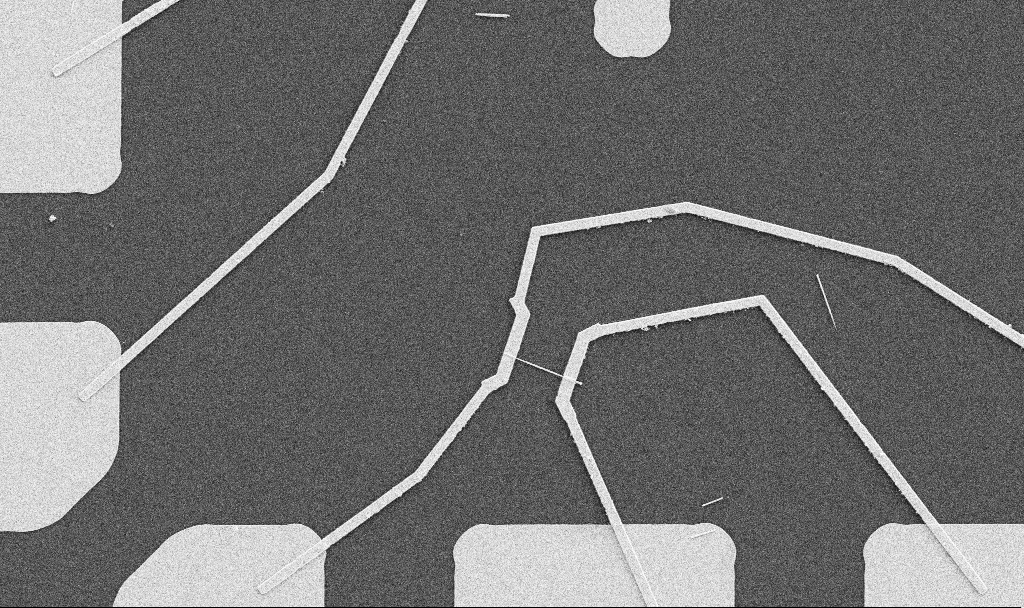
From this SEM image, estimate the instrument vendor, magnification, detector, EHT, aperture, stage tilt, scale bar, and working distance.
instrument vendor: Zeiss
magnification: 5 K X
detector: SE2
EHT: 5 kV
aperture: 30 µm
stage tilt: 0°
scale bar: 10000 nm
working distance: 10.7 mm